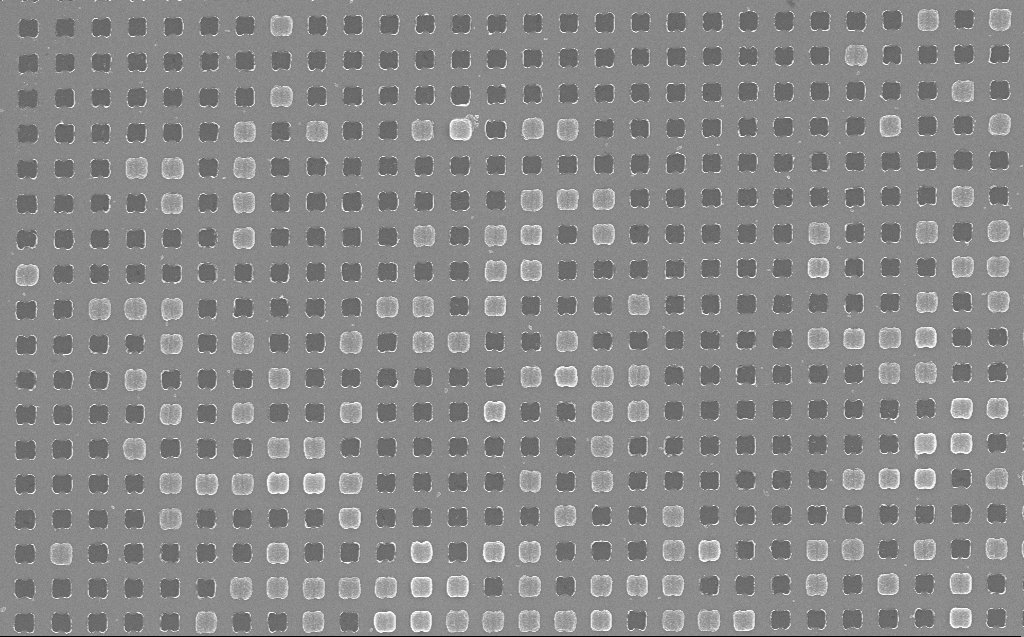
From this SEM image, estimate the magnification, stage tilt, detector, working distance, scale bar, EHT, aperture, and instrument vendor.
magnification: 26.7 K X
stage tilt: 0°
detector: InLens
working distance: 5 mm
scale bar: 2000 nm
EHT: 10 kV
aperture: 30 µm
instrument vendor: Zeiss